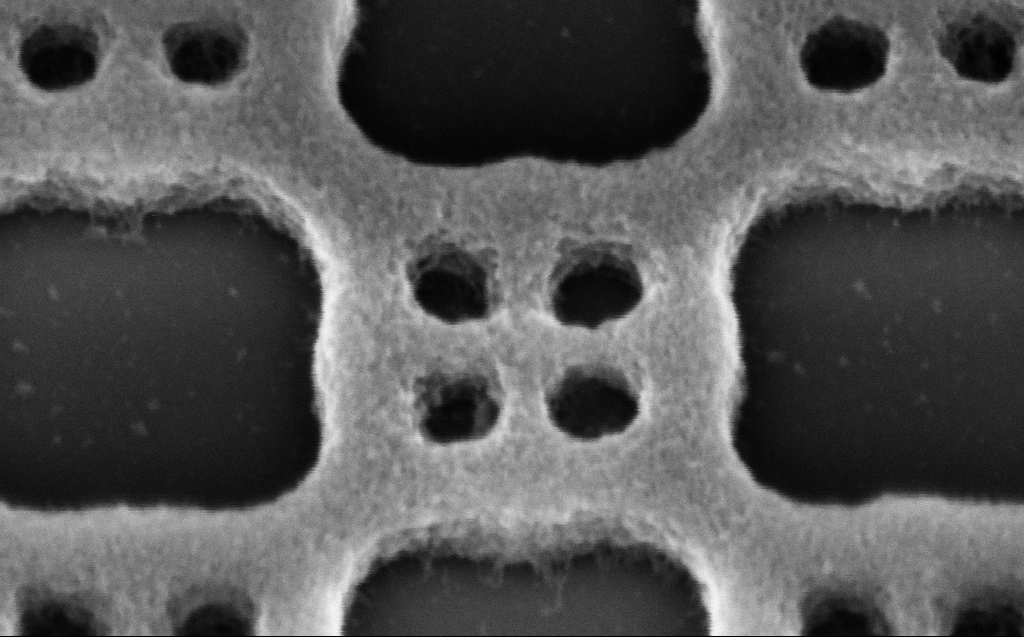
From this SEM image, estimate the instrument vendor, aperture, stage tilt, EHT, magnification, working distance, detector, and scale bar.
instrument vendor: Zeiss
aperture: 30 µm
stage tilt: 30°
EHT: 3 kV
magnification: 289.01 K X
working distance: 5 mm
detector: InLens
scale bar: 200 nm